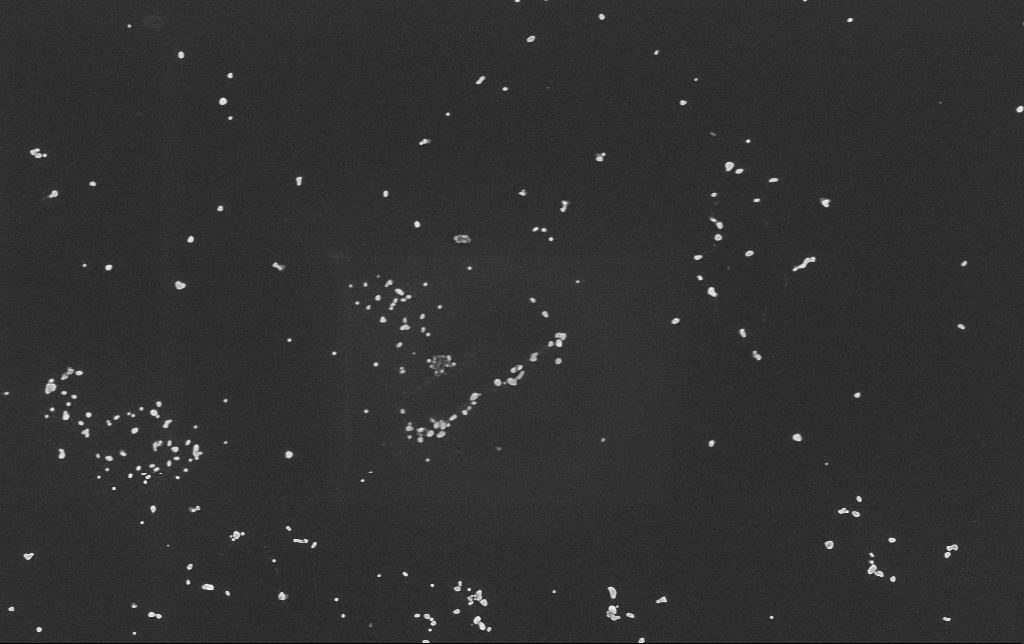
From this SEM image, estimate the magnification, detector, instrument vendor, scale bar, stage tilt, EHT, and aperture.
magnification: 50 K X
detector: InLens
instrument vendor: Zeiss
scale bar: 1000 nm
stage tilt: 0°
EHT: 10 kV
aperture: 30 µm